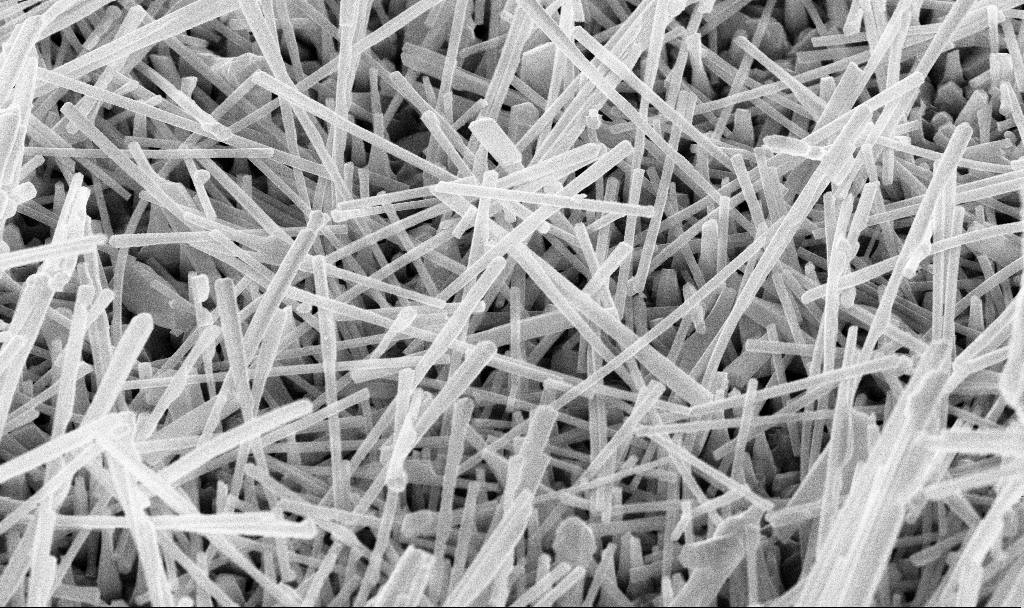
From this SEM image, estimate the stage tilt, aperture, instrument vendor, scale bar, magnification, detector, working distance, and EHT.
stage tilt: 45°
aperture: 30 µm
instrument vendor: Zeiss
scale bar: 2000 nm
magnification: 34.27 K X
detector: InLens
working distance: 7 mm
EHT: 10 kV